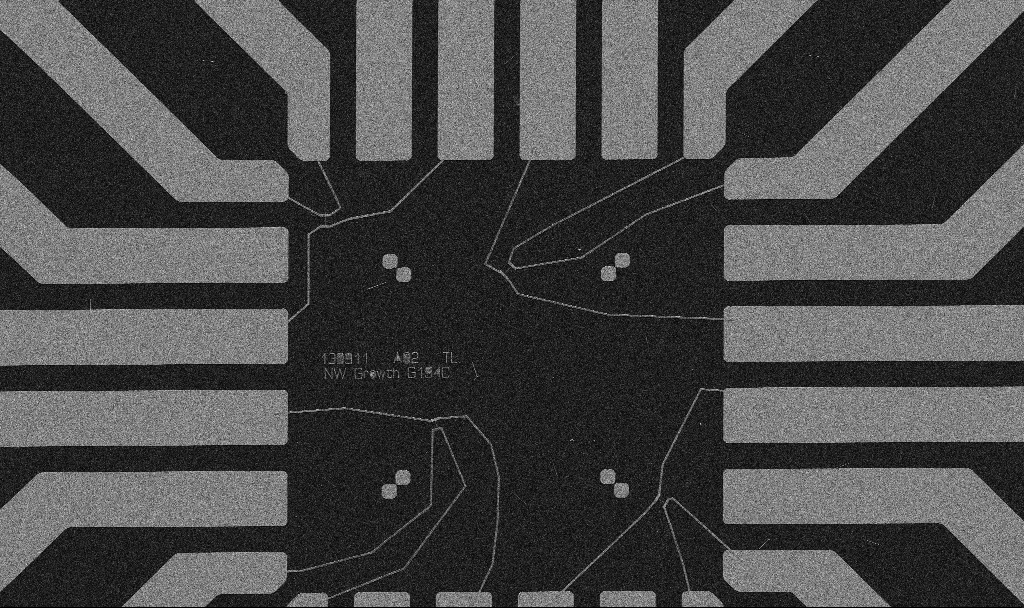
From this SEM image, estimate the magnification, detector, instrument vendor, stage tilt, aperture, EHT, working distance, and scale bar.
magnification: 1 K X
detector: SE2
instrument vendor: Zeiss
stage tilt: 0°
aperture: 30 µm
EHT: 5 kV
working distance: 10.7 mm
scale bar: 20000 nm